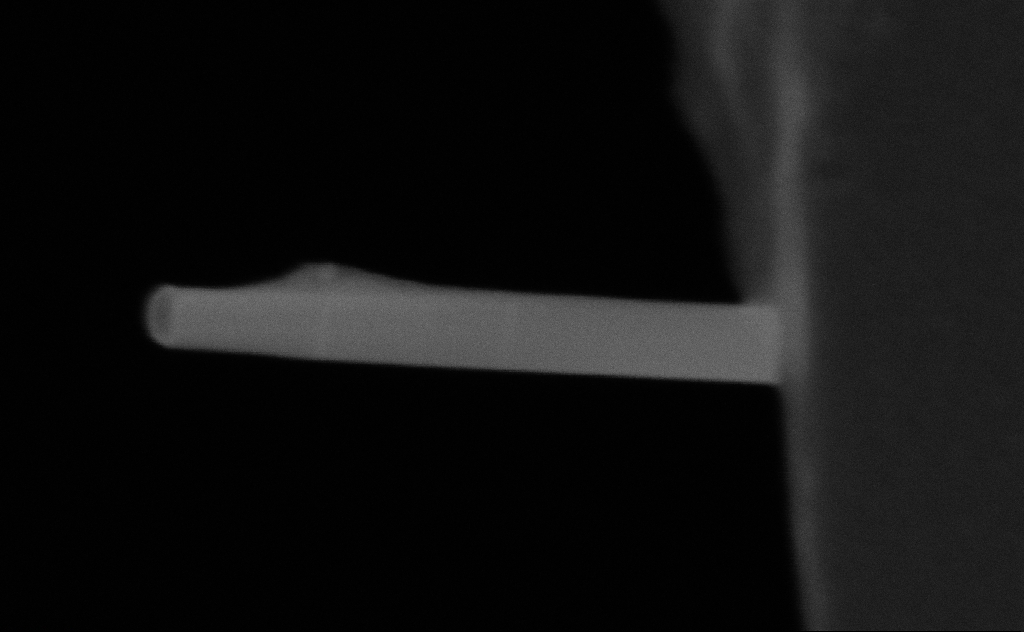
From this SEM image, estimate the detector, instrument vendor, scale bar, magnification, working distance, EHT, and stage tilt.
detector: SE2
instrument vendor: Zeiss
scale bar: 100 nm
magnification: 400.51 K X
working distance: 9 mm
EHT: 20 kV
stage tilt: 0°